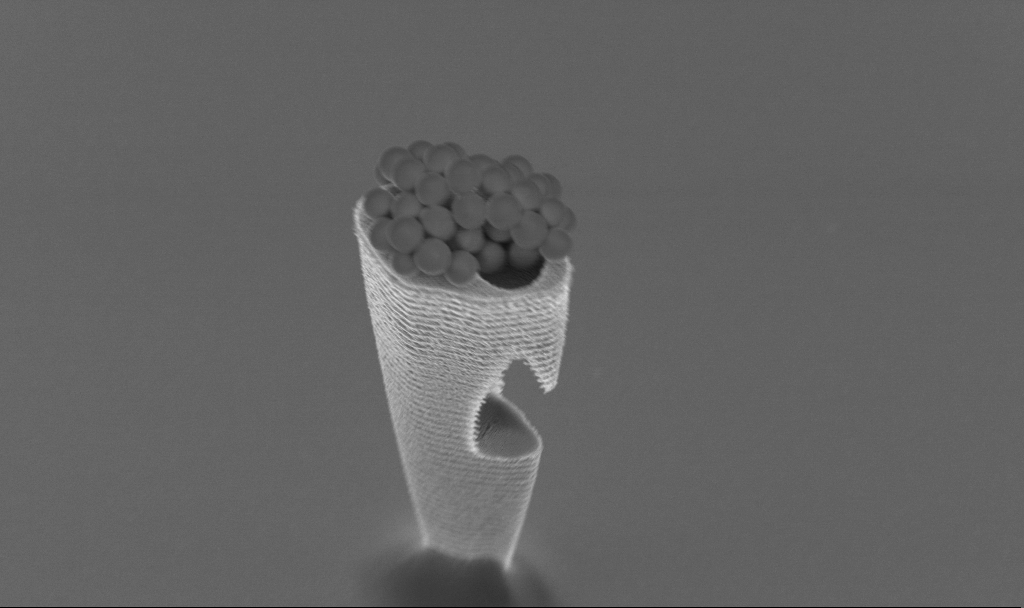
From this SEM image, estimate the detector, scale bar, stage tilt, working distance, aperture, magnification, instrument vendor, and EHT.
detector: InLens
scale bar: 1000 nm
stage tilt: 20°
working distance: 3.1 mm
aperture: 30 µm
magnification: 21.36 K X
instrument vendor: Zeiss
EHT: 3 kV